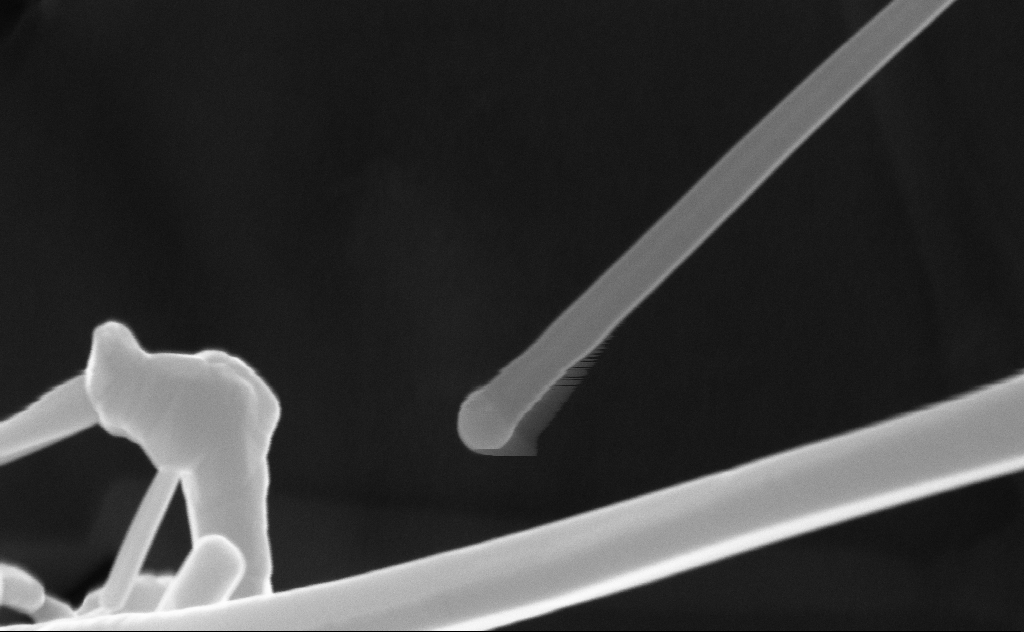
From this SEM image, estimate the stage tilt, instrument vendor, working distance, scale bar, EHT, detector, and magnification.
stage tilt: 0°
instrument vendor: Zeiss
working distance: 6 mm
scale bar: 200 nm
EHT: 10 kV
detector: InLens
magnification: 172.85 K X